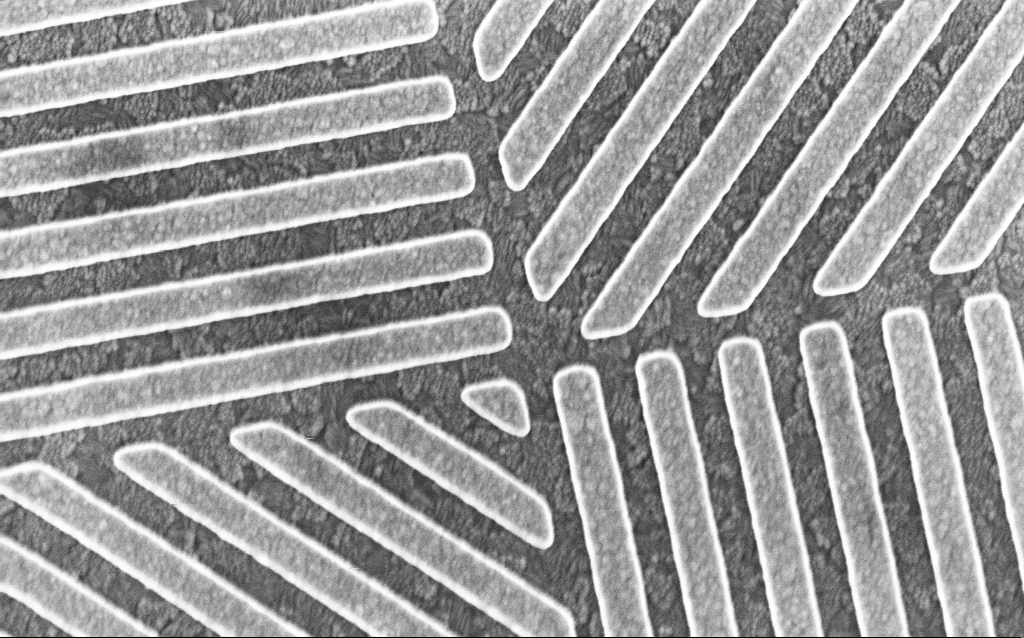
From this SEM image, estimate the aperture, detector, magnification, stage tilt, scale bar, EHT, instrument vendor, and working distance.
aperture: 30 µm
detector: InLens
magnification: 77.5 K X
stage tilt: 0°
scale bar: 200 nm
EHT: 3 kV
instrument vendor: Zeiss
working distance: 4.5 mm